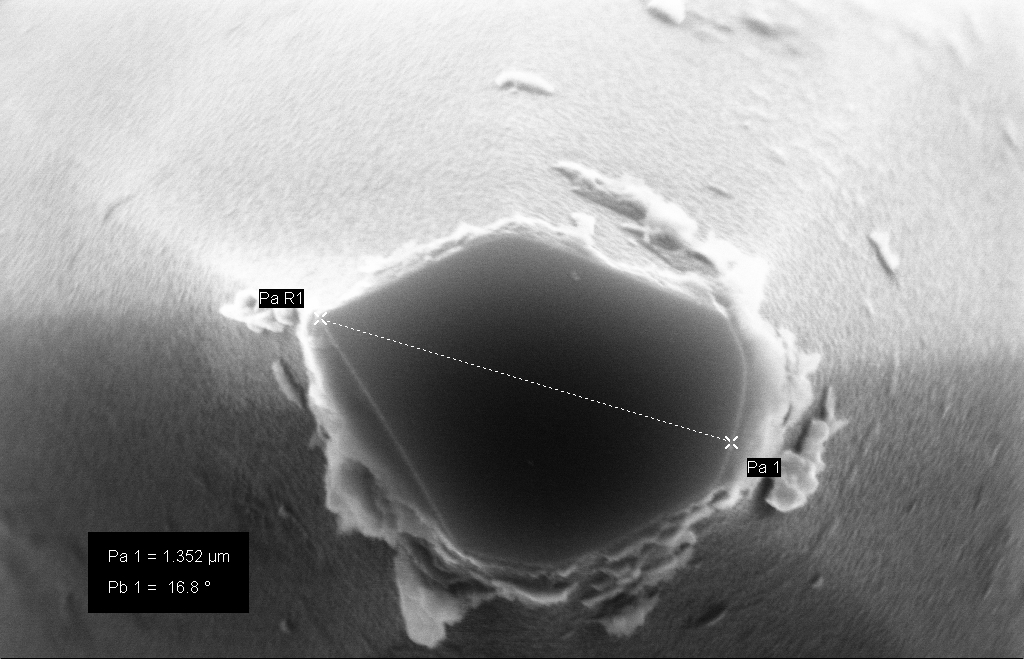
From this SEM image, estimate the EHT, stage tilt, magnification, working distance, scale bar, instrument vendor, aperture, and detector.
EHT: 10 kV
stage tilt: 0°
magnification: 116.61 K X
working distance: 8 mm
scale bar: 100 nm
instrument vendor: Zeiss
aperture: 30 µm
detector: InLens